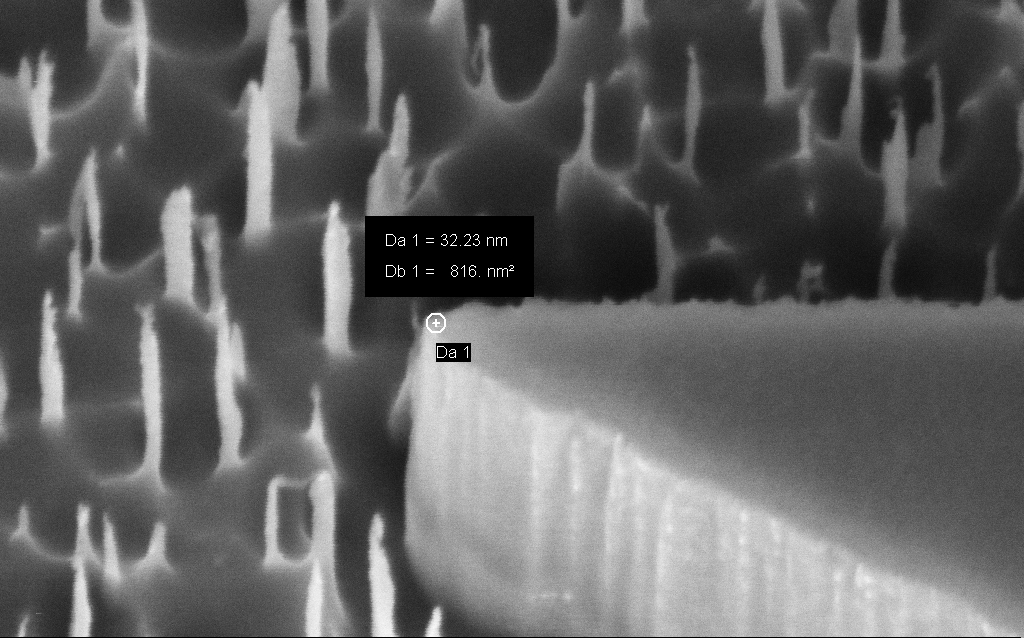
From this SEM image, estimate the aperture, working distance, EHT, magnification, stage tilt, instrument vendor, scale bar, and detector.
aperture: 30 µm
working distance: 8 mm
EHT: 3 kV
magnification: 227.84 K X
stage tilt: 45°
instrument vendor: Zeiss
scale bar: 200 nm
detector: InLens